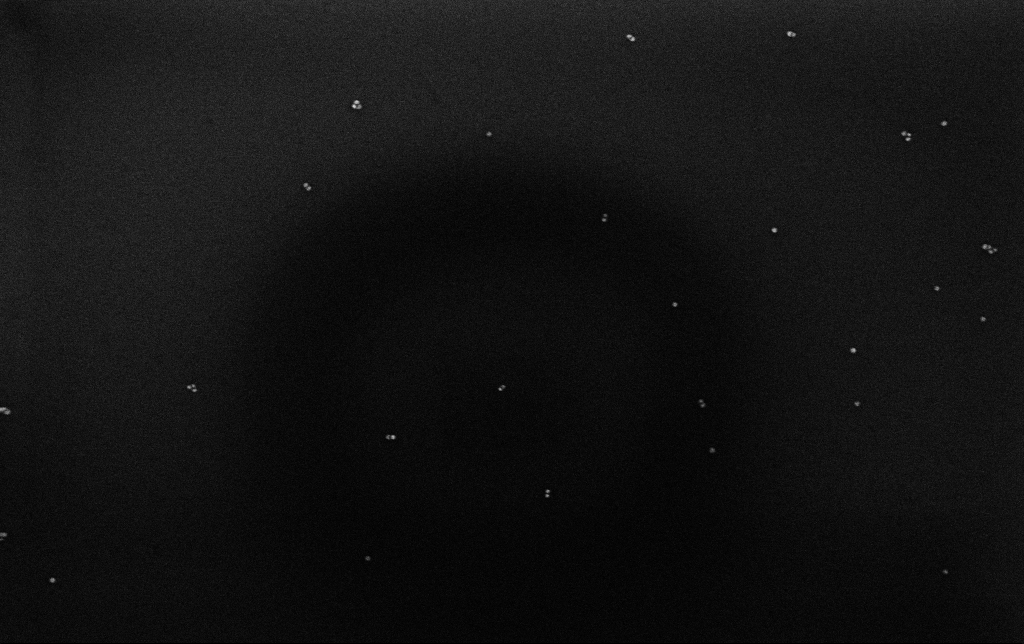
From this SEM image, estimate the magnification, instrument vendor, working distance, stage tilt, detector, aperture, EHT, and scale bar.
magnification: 100 K X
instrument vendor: Zeiss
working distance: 3.2 mm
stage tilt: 0°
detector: InLens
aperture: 30 µm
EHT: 10 kV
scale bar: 200 nm